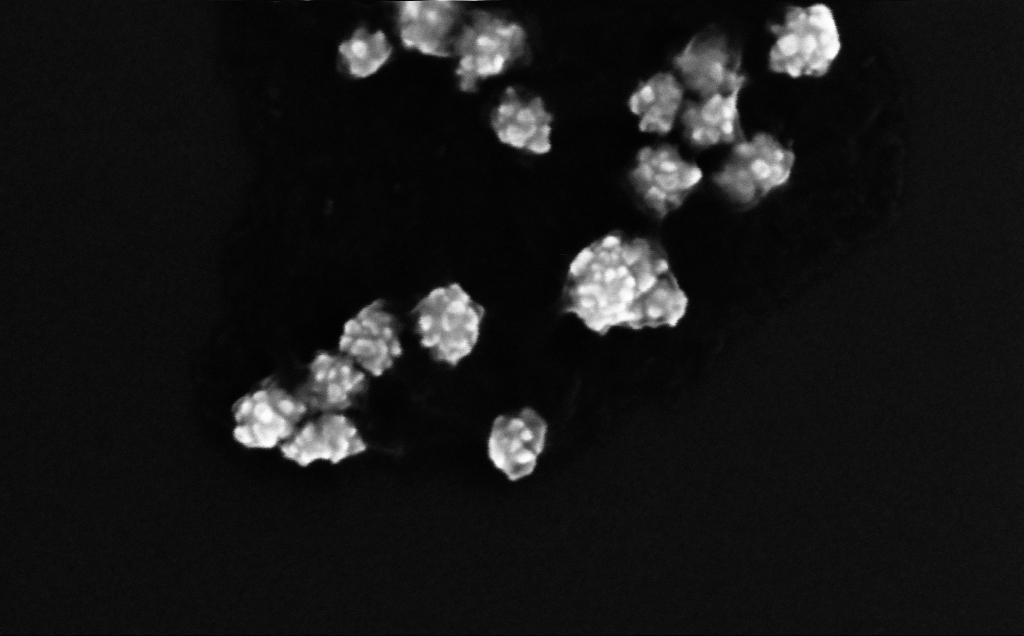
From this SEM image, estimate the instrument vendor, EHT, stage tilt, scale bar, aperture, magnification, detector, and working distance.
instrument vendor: Zeiss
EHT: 10 kV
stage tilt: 0°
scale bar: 100 nm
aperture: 30 µm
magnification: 373.91 K X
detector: InLens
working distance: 4 mm